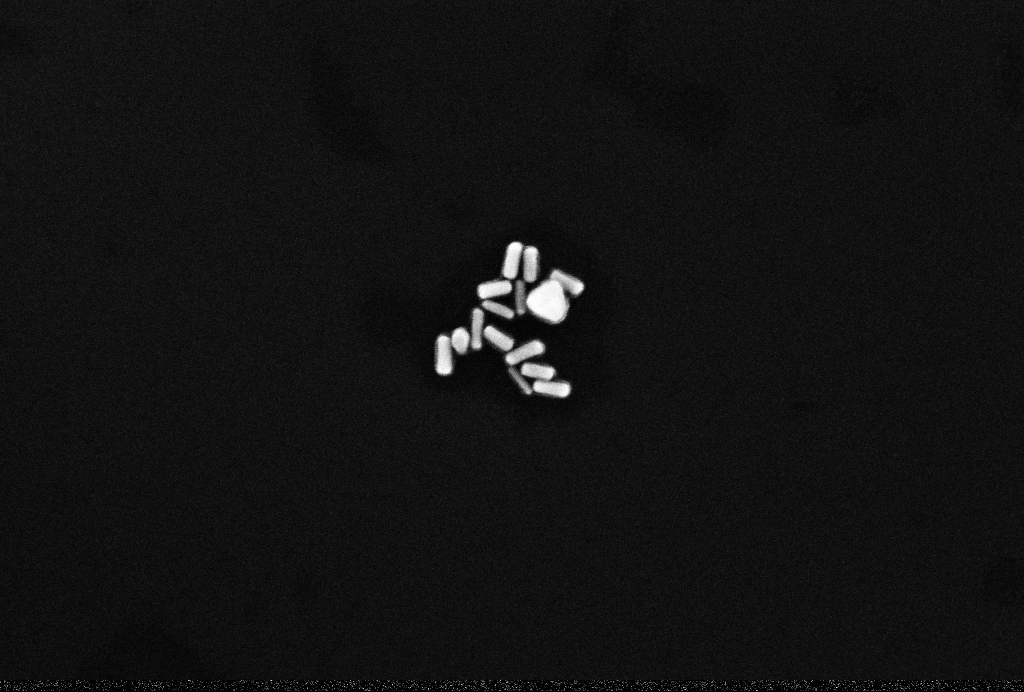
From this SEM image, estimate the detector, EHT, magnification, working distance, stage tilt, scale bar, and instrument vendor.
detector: InLens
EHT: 10 kV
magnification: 200 K X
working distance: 3.2 mm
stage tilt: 0°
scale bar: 200 nm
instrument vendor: Zeiss